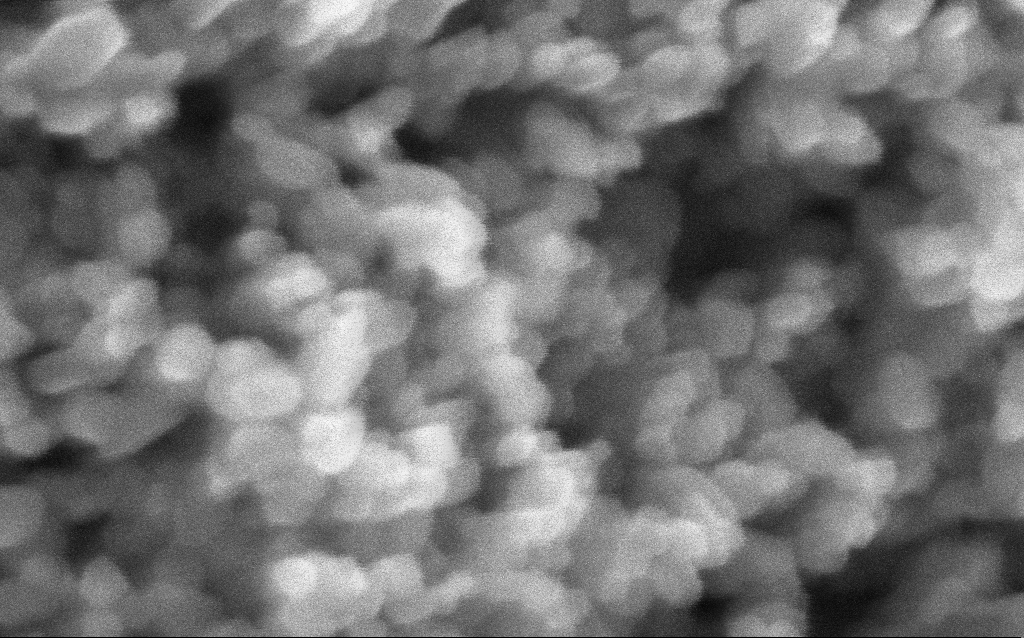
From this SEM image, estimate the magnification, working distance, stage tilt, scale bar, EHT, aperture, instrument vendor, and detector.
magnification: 716 K X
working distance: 2.9 mm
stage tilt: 0°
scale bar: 100 nm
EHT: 5 kV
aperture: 30 µm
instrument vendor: Zeiss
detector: InLens